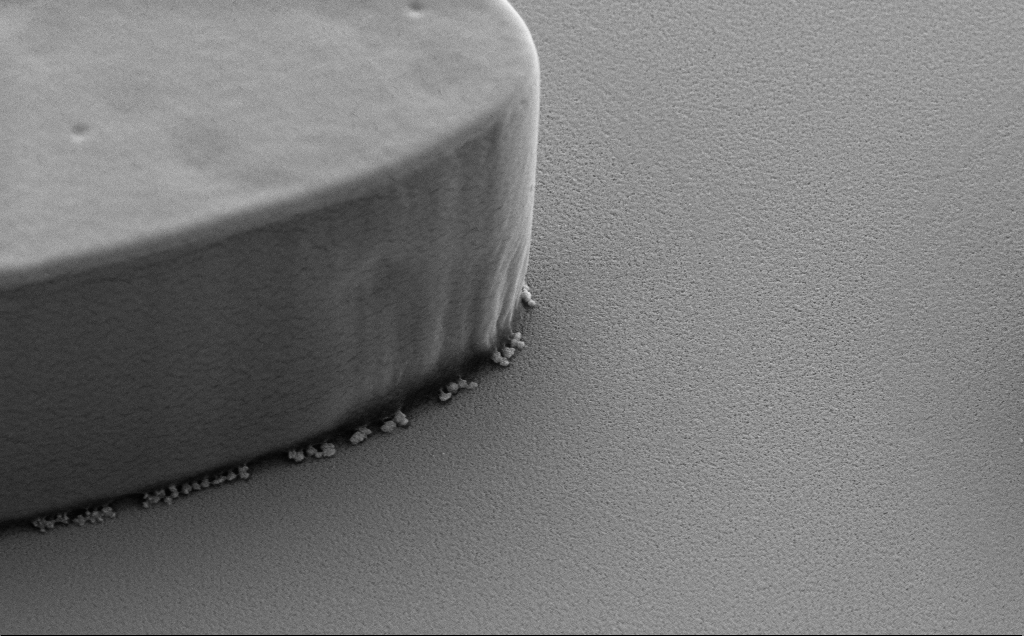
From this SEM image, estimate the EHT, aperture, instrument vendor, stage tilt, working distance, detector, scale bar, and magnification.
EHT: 5 kV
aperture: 30 µm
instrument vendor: Zeiss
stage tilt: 40°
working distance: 8 mm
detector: SE2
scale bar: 1000 nm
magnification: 19.3 K X